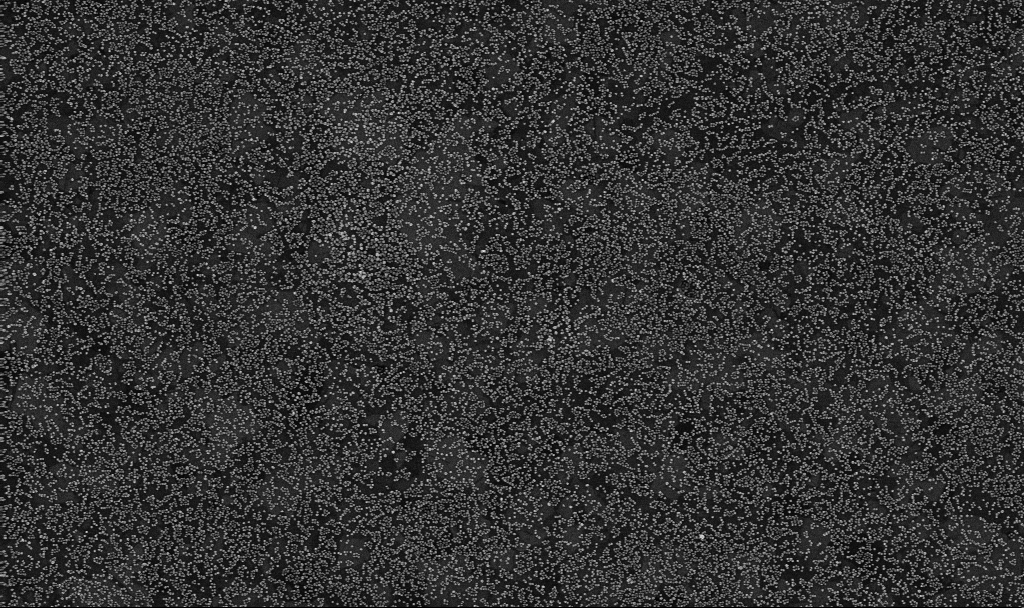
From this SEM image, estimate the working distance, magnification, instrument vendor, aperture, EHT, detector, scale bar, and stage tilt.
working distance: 3.3 mm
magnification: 50 K X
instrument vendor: Zeiss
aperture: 30 µm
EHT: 10 kV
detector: InLens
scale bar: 1000 nm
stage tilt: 0°